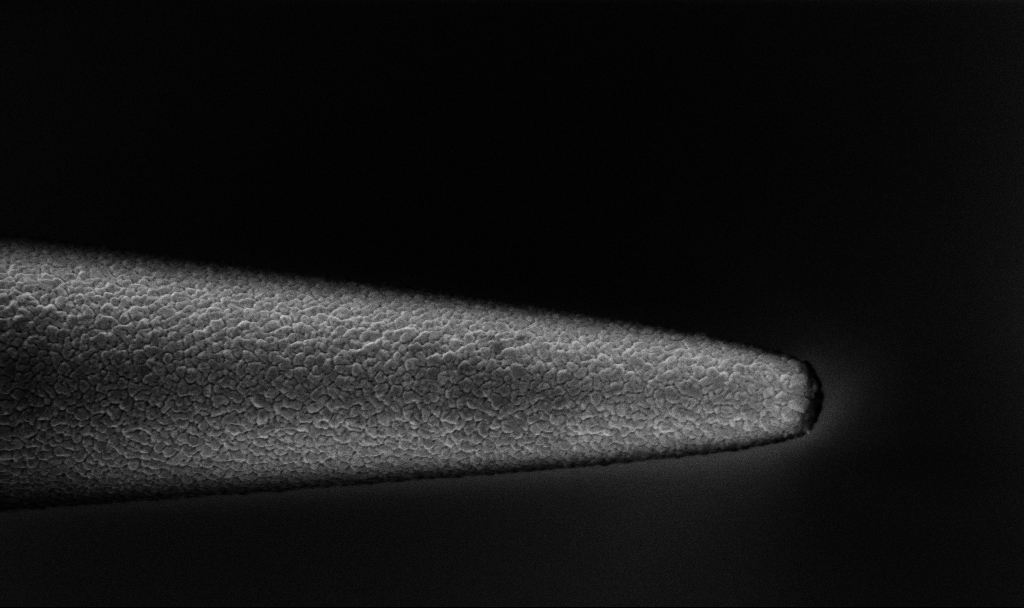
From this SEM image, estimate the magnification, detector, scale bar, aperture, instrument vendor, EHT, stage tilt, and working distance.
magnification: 50 K X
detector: InLens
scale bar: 1000 nm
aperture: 30 µm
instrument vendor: Zeiss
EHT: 1.5 kV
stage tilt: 45°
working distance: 3.3 mm